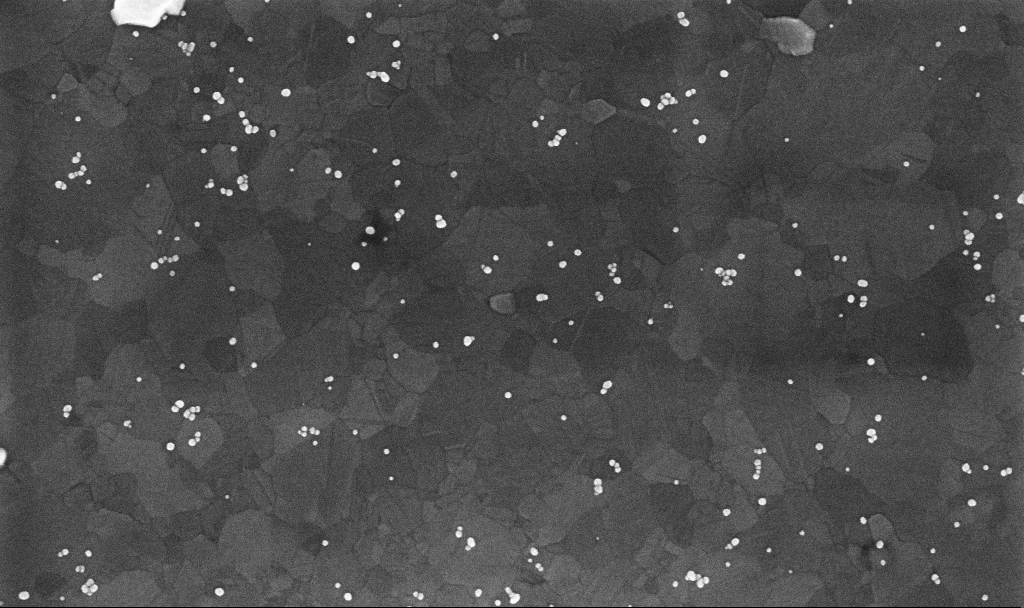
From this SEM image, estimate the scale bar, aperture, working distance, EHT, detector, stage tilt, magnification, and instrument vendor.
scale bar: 200 nm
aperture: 30 µm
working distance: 3.4 mm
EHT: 10 kV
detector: InLens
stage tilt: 0°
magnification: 100.84 K X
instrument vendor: Zeiss